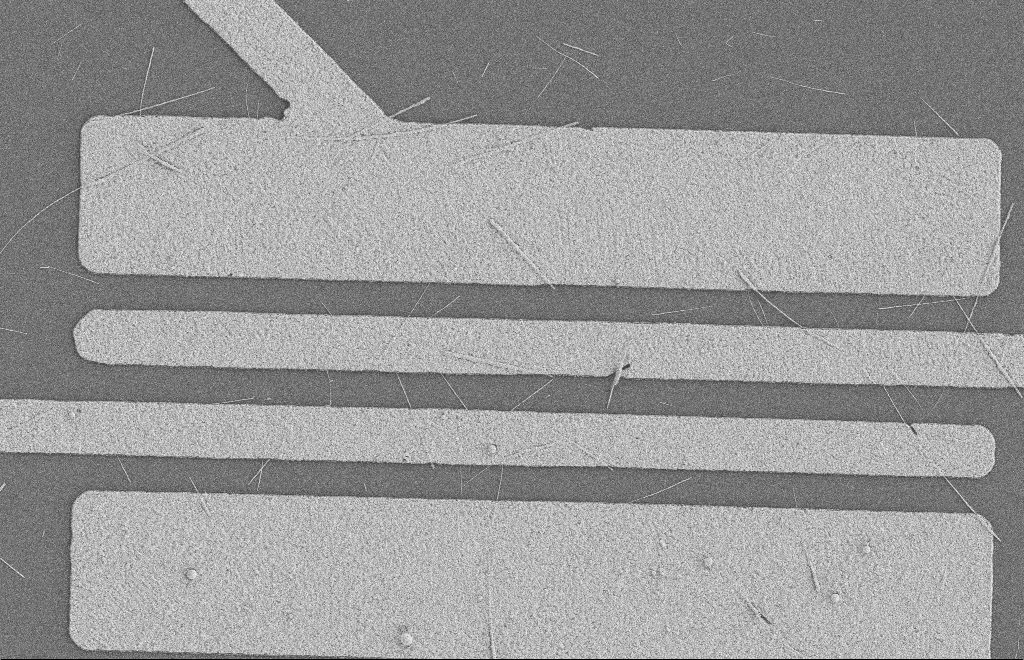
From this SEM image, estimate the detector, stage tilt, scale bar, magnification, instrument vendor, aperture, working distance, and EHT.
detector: SE2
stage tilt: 0°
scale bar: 2000 nm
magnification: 5.54 K X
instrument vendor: Zeiss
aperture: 20 µm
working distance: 8 mm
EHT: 2 kV